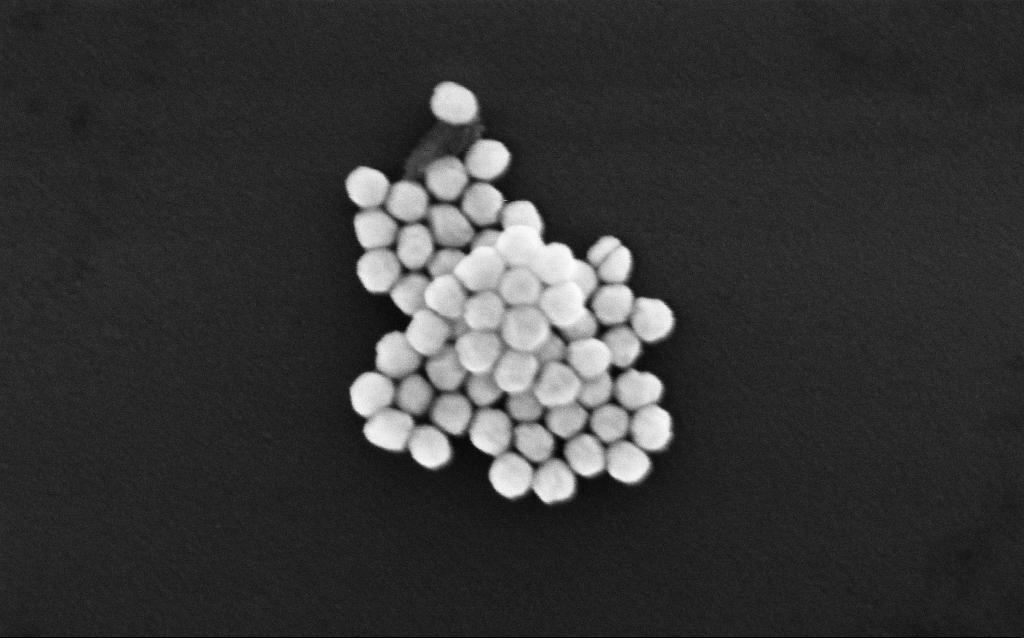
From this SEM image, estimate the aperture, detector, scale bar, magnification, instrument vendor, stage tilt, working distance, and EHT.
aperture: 30 µm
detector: InLens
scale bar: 200 nm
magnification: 259.3 K X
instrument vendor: Zeiss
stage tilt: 0°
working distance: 3.2 mm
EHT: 8 kV